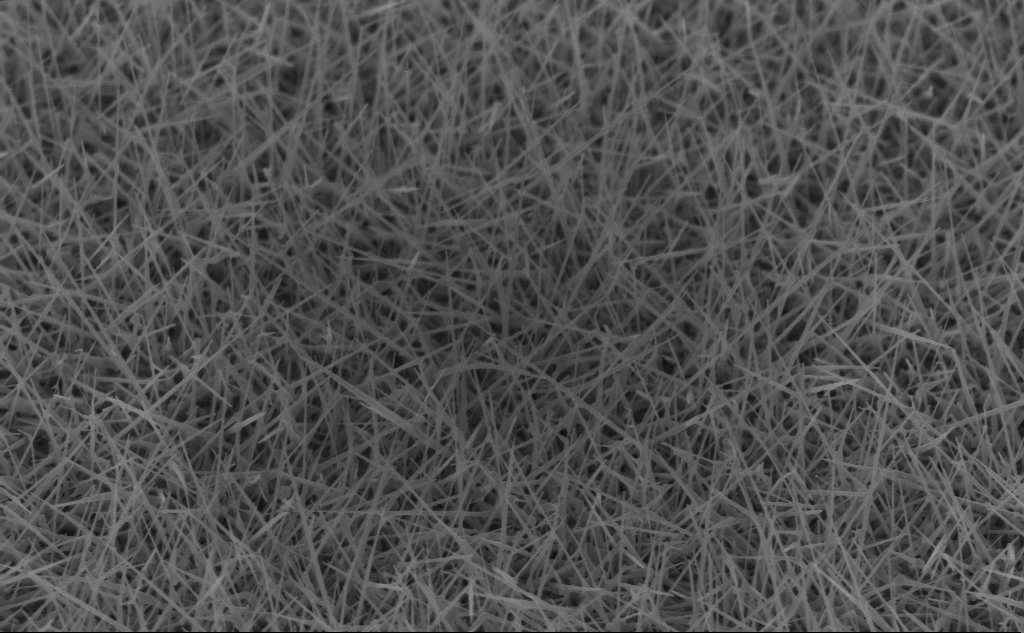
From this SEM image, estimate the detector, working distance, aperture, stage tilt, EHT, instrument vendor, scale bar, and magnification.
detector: InLens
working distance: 4 mm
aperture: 30 µm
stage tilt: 45°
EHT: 5 kV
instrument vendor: Zeiss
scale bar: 1000 nm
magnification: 20 K X